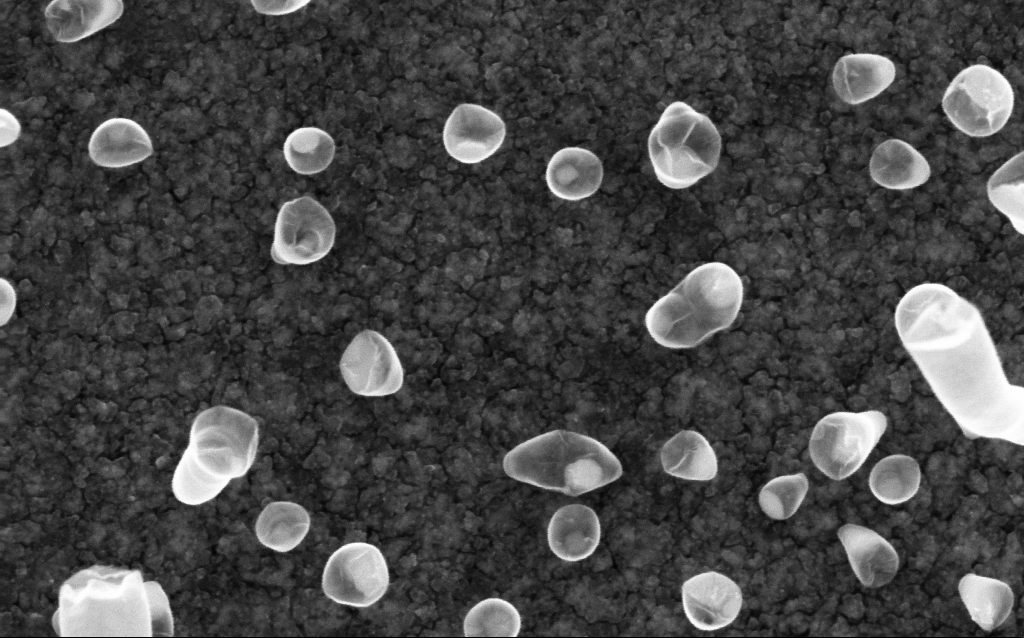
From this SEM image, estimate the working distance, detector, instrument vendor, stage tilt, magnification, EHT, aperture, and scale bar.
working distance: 2 mm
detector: InLens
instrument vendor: Zeiss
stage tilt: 0°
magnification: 200 K X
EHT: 5 kV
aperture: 30 µm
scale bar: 100 nm